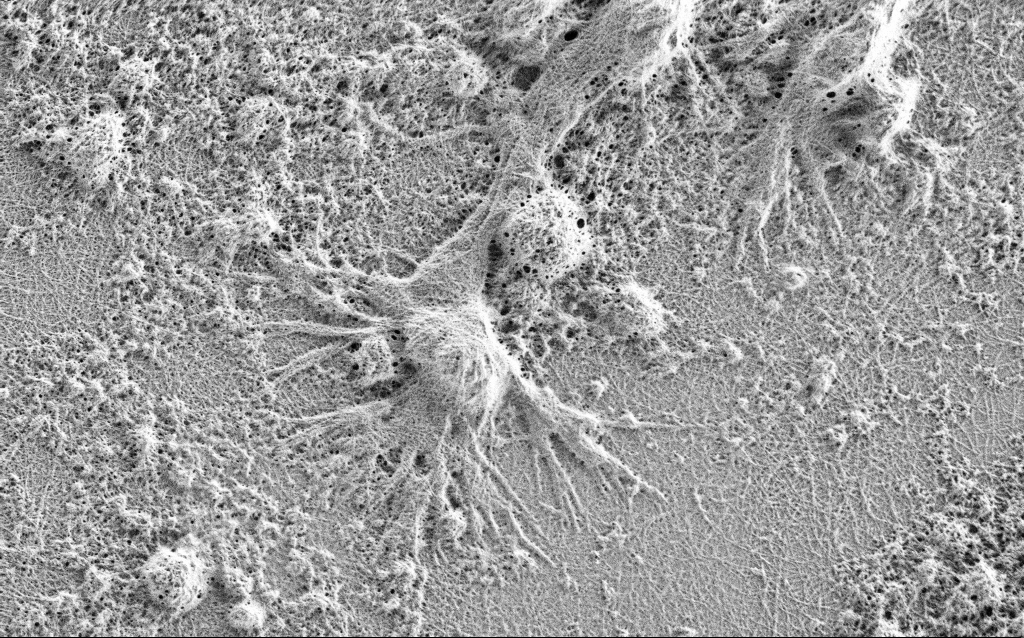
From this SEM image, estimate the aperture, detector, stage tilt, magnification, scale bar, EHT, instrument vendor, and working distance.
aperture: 30 µm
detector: SE2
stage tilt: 0°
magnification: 10 K X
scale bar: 2000 nm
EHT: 0.9 kV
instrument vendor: Zeiss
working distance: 4 mm